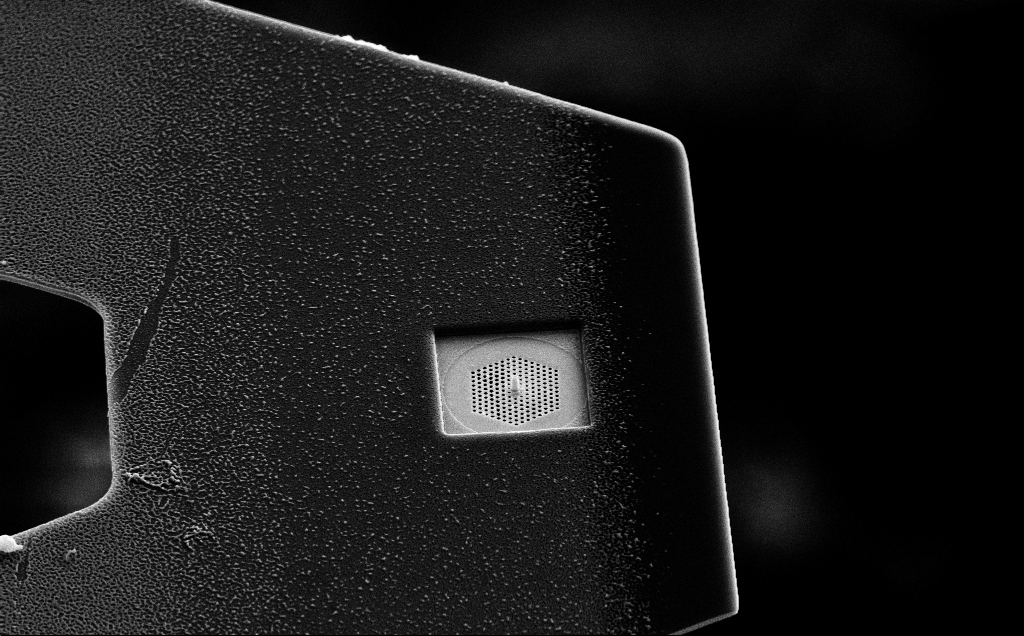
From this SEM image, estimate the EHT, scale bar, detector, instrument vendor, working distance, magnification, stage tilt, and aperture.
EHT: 10 kV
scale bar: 2000 nm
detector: SE2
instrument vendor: Zeiss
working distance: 11 mm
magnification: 8.75 K X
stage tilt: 45°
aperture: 30 µm